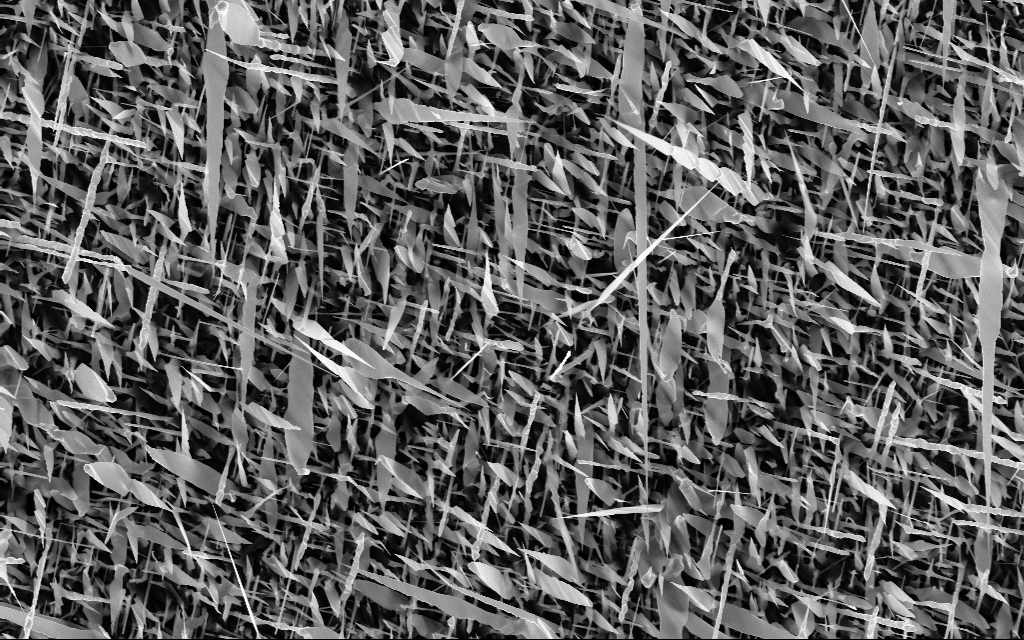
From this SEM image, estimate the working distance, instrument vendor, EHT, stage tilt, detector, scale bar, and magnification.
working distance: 6 mm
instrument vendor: Zeiss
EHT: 10 kV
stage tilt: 0°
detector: InLens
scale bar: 2000 nm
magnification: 10 K X